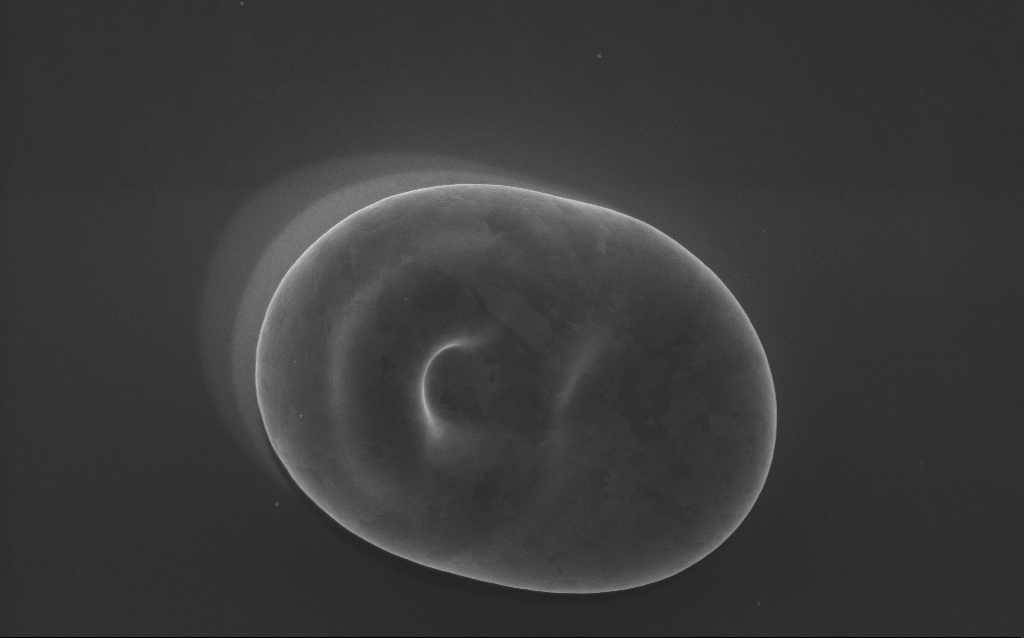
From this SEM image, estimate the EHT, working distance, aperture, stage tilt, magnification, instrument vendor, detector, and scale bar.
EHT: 5 kV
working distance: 3 mm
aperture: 30 µm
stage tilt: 0°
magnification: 38 K X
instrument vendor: Zeiss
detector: InLens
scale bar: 1000 nm